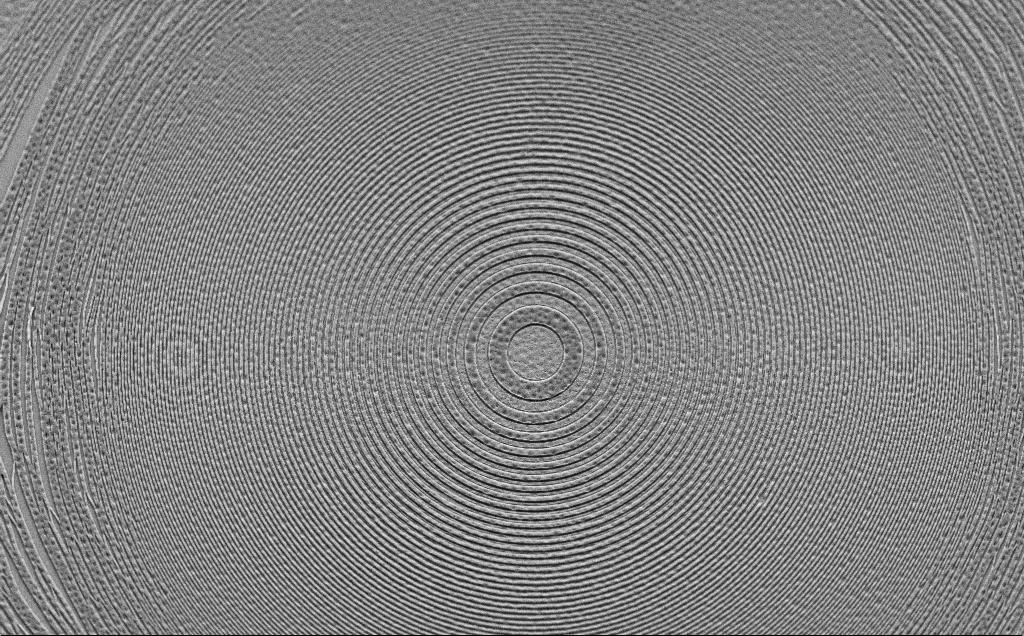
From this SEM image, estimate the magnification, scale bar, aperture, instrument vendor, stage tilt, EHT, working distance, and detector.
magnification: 2.87 K X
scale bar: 10000 nm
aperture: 30 µm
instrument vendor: Zeiss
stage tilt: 45°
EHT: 5 kV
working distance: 6 mm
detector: SE2